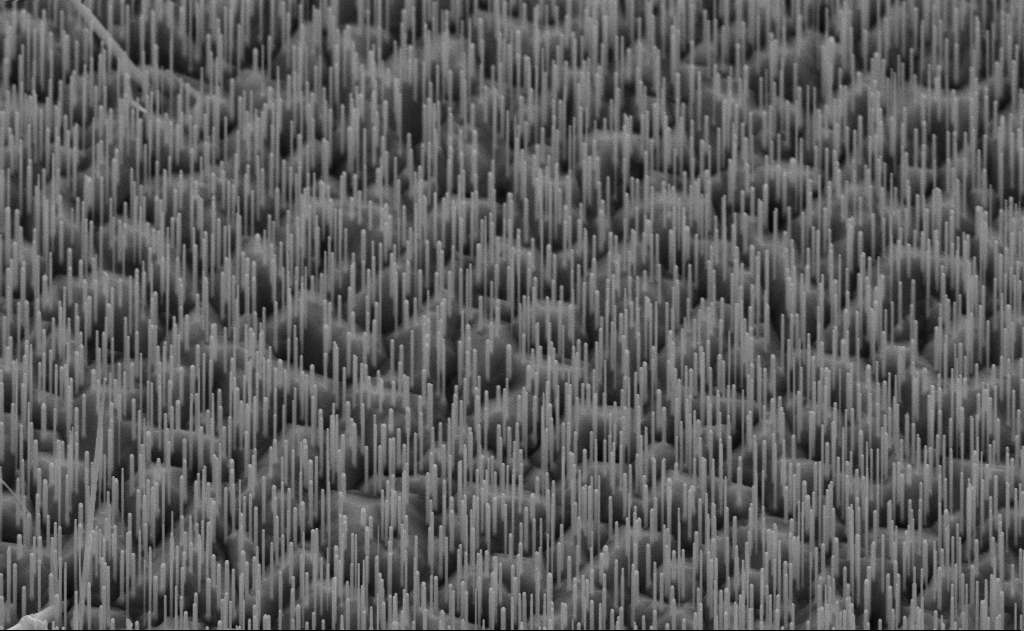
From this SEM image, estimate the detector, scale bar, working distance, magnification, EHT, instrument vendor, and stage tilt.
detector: SE2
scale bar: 2000 nm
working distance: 10 mm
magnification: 20 K X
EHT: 10 kV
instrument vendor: Zeiss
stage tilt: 45°